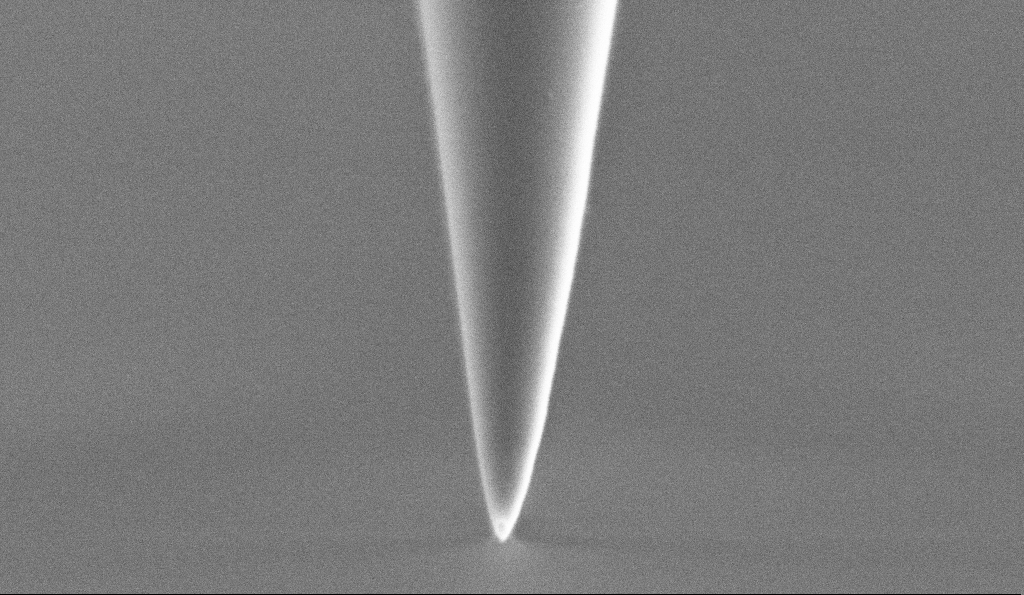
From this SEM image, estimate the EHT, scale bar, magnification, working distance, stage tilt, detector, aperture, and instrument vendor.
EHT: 1 kV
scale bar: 200 nm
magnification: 75 K X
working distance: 6.6 mm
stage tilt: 45°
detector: SE2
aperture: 30 µm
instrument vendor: Zeiss